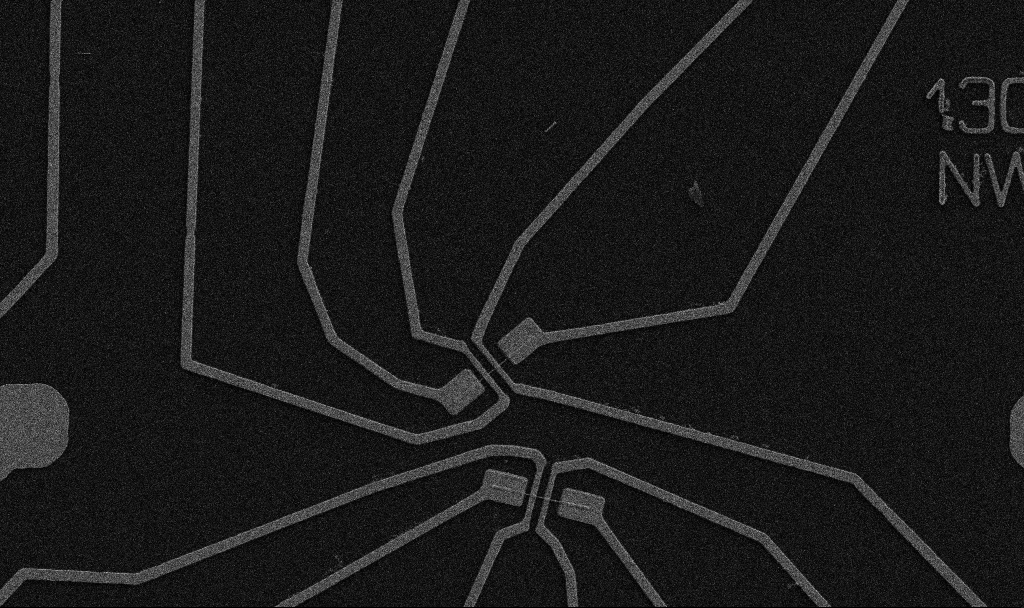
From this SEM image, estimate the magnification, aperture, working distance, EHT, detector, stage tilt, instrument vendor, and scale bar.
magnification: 5 K X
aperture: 30 µm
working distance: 10.7 mm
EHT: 5 kV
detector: SE2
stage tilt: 0°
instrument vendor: Zeiss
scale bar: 10000 nm